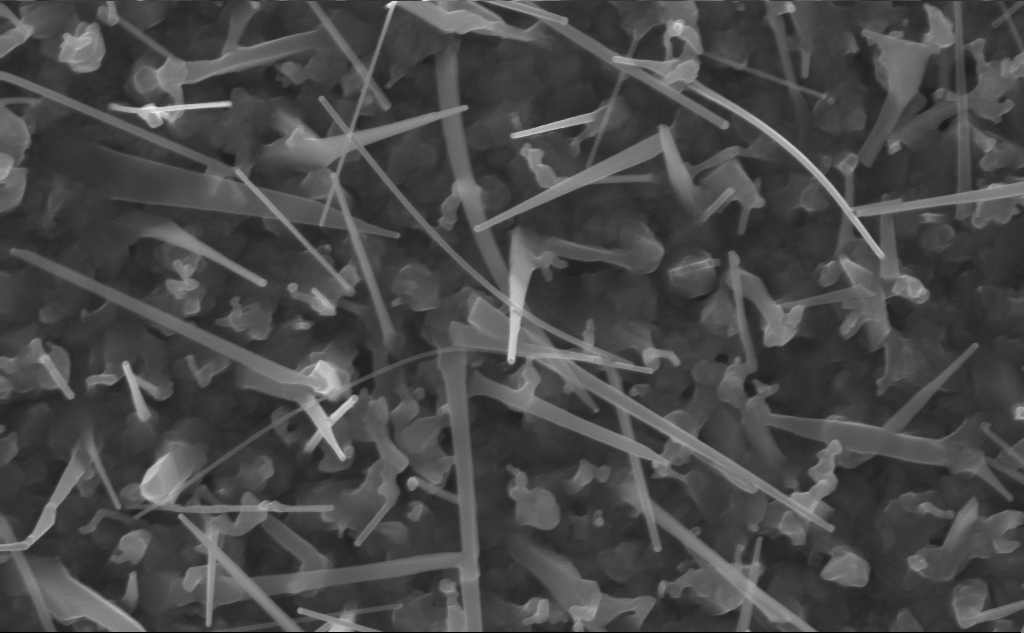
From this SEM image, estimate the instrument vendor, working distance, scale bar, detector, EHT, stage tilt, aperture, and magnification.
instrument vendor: Zeiss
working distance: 5 mm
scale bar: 200 nm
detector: InLens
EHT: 10 kV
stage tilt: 0°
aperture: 30 µm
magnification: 80 K X